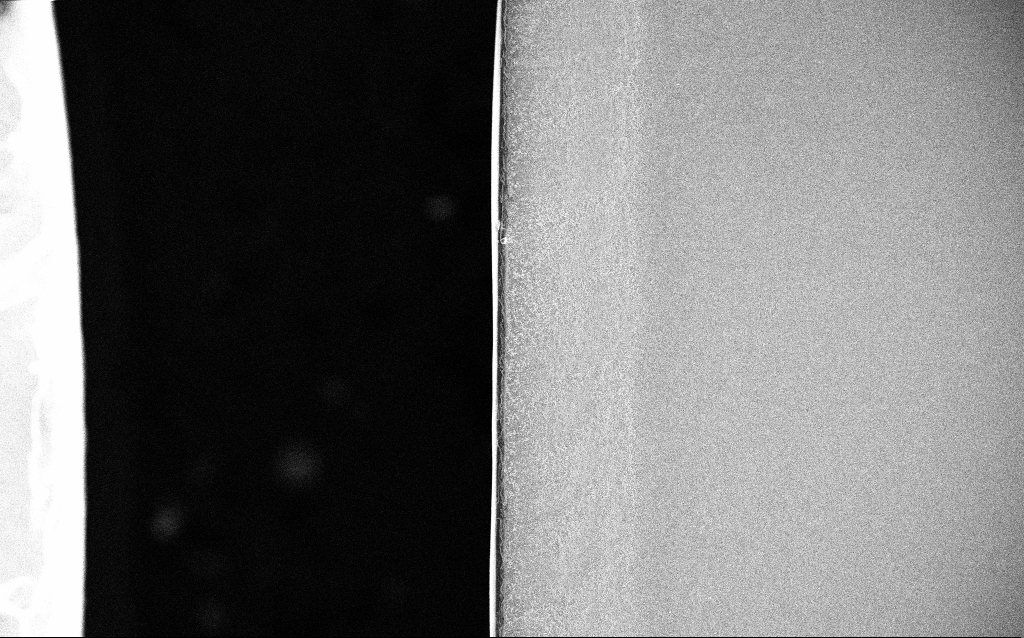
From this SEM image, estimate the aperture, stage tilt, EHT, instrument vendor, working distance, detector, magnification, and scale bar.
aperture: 30 µm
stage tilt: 0°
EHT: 20 kV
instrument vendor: Zeiss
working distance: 1.8 mm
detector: InLens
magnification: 0.25 K X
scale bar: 100000 nm